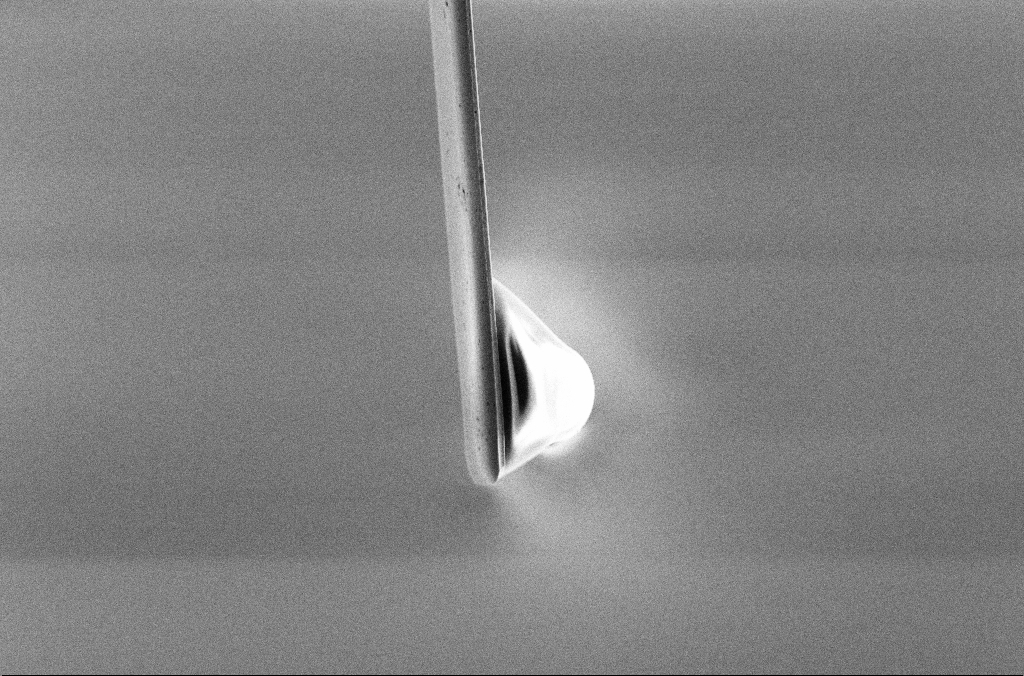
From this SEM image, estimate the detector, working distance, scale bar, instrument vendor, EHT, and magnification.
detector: SE2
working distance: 5.3 mm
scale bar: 10000 nm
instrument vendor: Zeiss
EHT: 5 kV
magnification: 2.5 K X